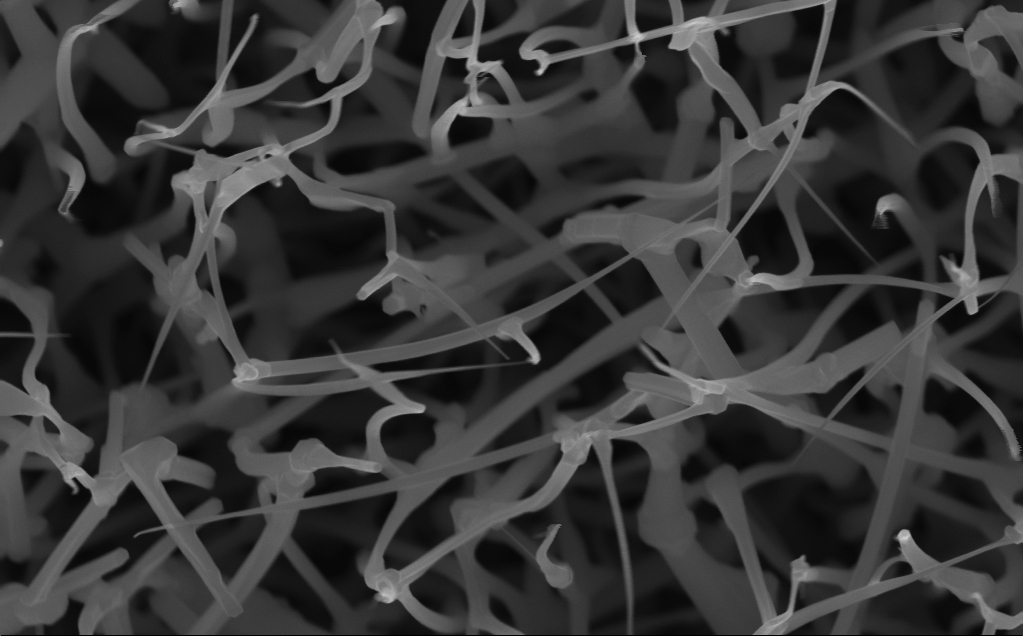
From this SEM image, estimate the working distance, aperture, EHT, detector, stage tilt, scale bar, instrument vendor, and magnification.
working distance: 5 mm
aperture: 30 µm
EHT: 10 kV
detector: InLens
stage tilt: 0°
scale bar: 200 nm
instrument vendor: Zeiss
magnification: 80 K X